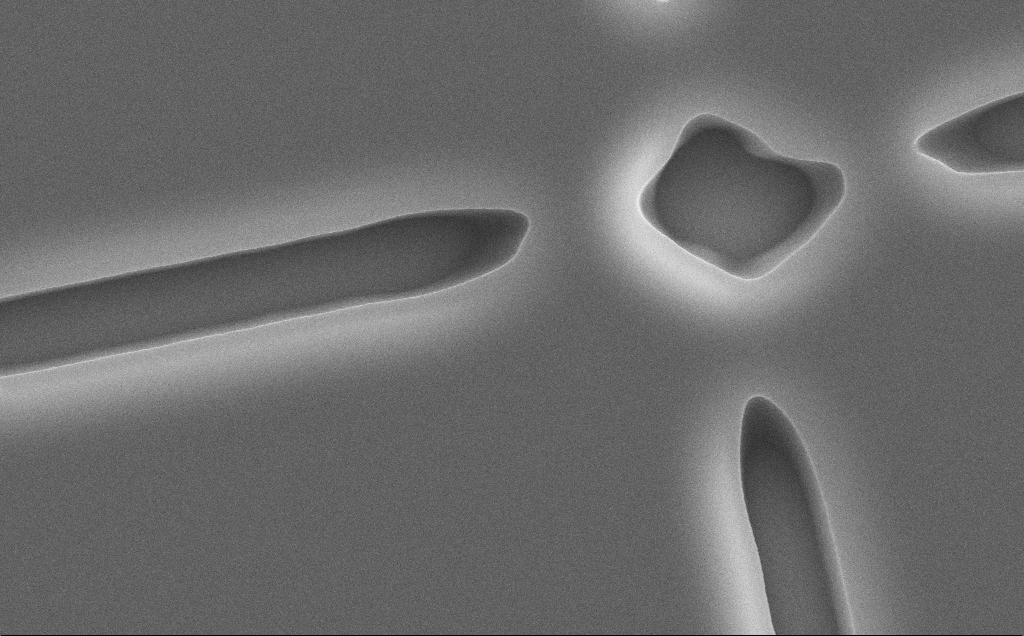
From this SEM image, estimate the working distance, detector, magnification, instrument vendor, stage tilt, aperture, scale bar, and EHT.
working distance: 12 mm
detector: SE2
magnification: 17.44 K X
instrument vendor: Zeiss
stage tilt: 0°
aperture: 30 µm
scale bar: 2000 nm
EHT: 10 kV